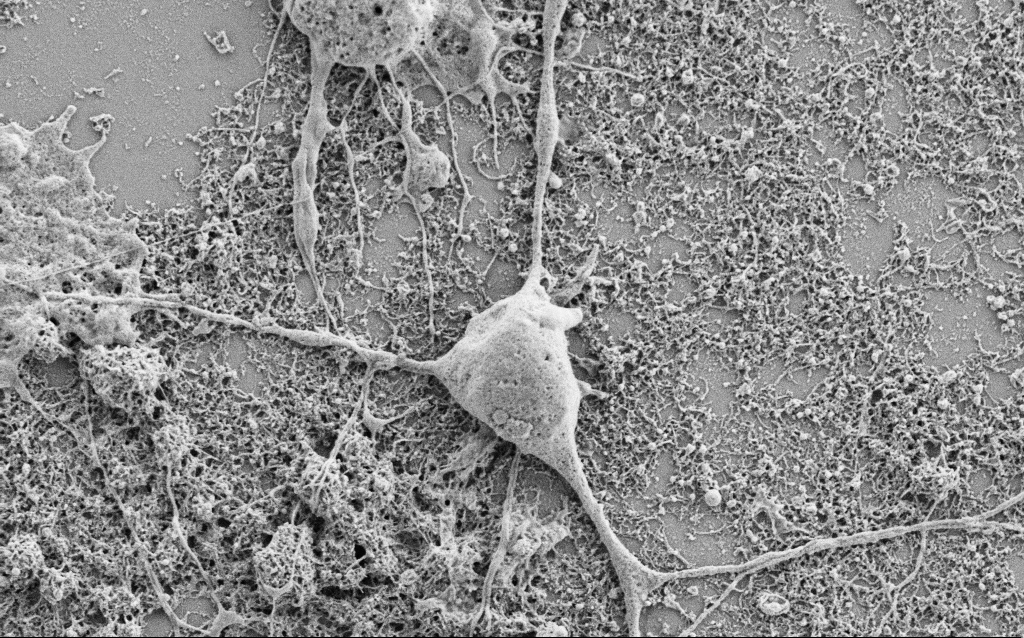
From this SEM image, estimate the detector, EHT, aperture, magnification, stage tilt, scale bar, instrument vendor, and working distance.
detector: SE2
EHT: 1.5 kV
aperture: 30 µm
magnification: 15 K X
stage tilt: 0°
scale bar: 2000 nm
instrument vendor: Zeiss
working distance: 6.8 mm